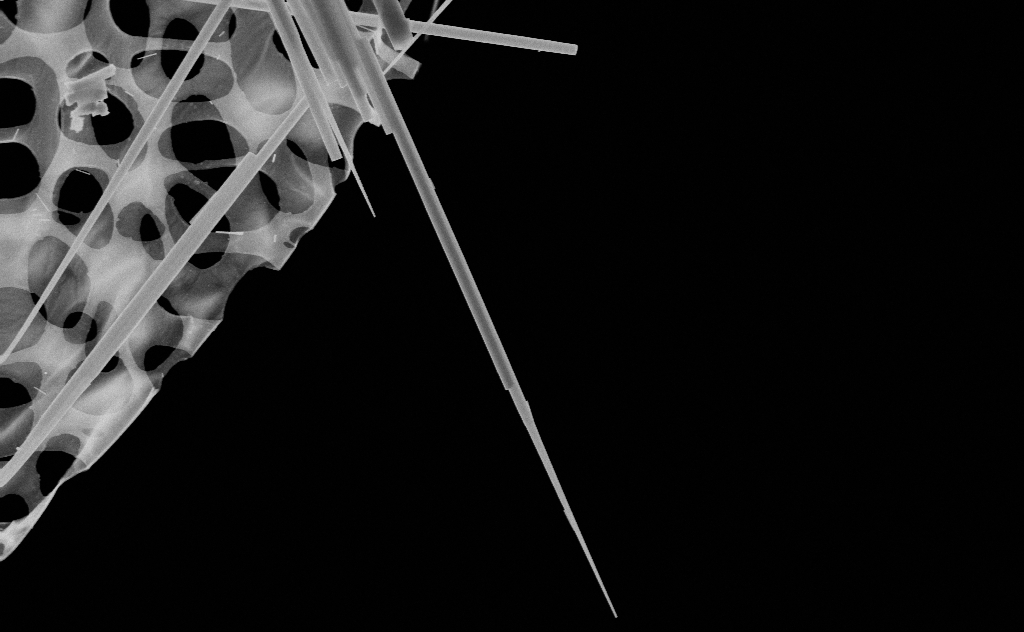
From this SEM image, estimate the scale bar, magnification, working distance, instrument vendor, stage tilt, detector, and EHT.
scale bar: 1000 nm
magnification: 31.54 K X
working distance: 10 mm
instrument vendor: Zeiss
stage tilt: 0°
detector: SE2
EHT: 20 kV